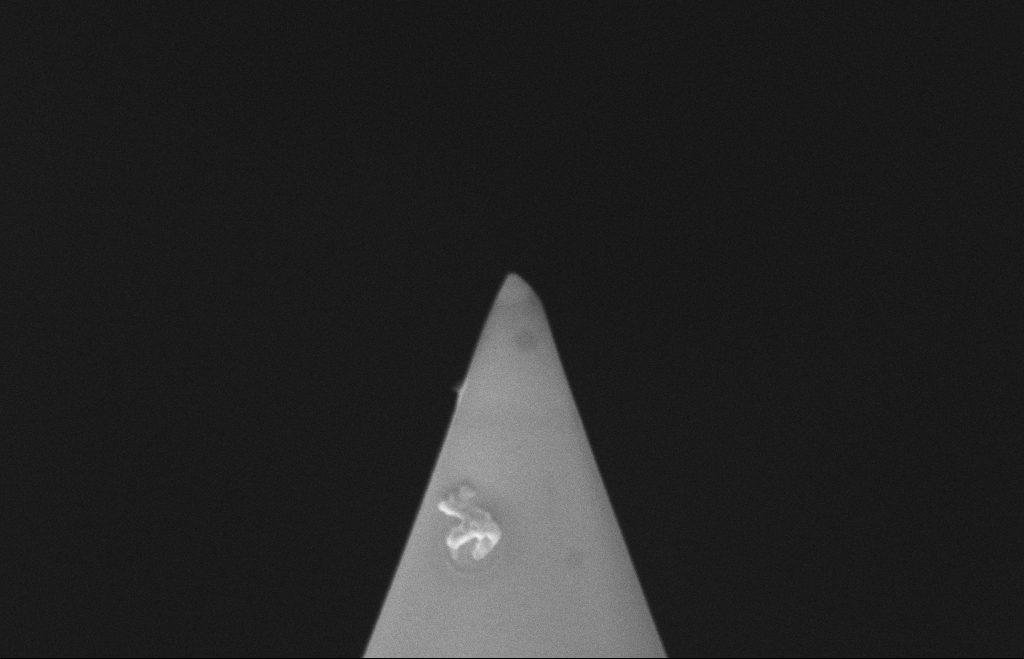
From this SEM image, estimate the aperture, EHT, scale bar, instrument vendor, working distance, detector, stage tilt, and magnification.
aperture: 30 µm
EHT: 10 kV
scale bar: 200 nm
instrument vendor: Zeiss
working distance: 8 mm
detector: InLens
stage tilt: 70°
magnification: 113.94 K X